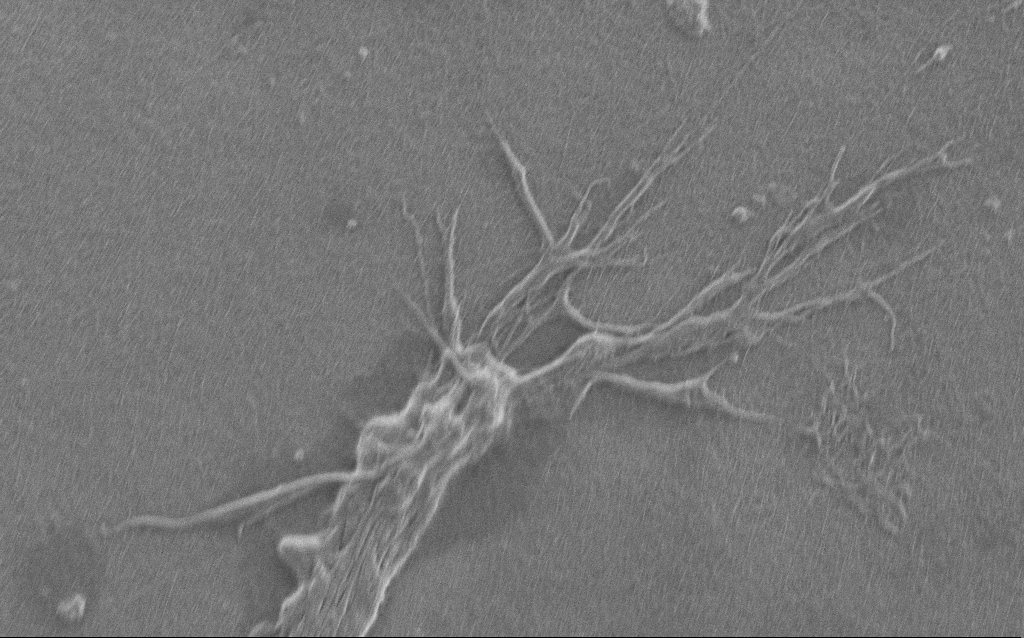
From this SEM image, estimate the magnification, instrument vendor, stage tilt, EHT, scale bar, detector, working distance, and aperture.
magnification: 7.5 K X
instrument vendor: Zeiss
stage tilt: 0°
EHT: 1 kV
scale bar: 2000 nm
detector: SE2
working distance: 6 mm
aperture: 30 µm